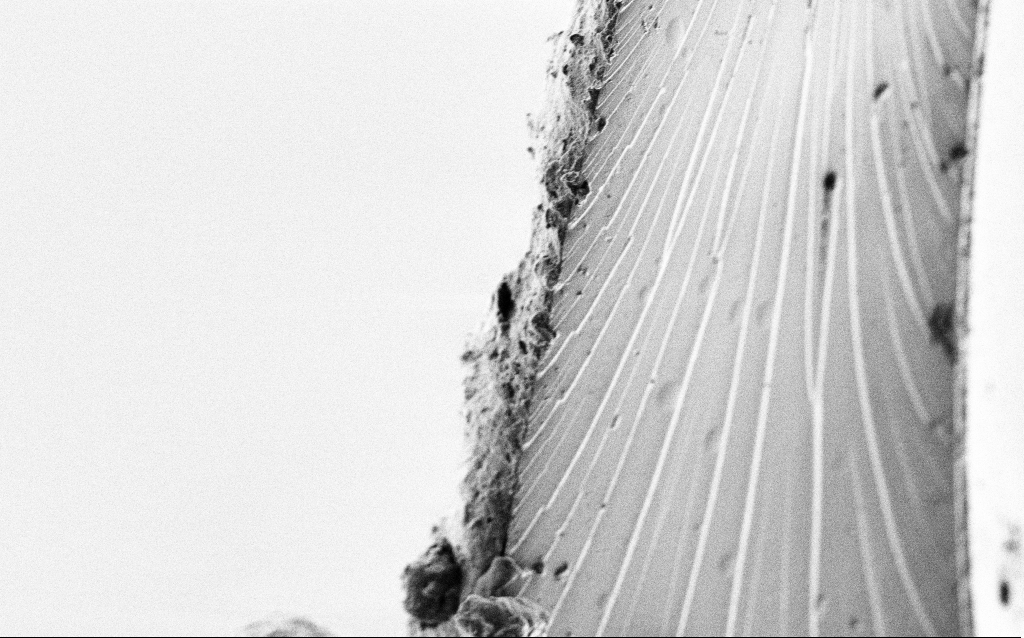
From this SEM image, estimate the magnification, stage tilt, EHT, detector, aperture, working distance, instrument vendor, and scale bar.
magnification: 10 K X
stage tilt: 45°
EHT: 1 kV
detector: SE2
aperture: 30 µm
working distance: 5 mm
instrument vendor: Zeiss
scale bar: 2000 nm